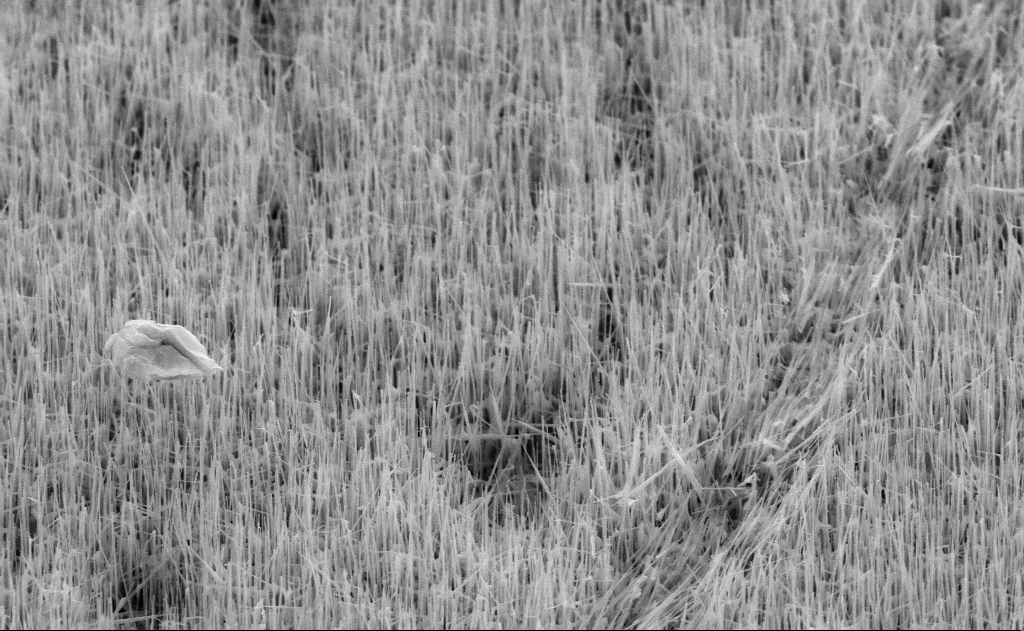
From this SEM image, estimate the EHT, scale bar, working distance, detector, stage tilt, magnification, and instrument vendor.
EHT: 10 kV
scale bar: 2000 nm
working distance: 11 mm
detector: SE2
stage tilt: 45°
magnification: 20 K X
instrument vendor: Zeiss